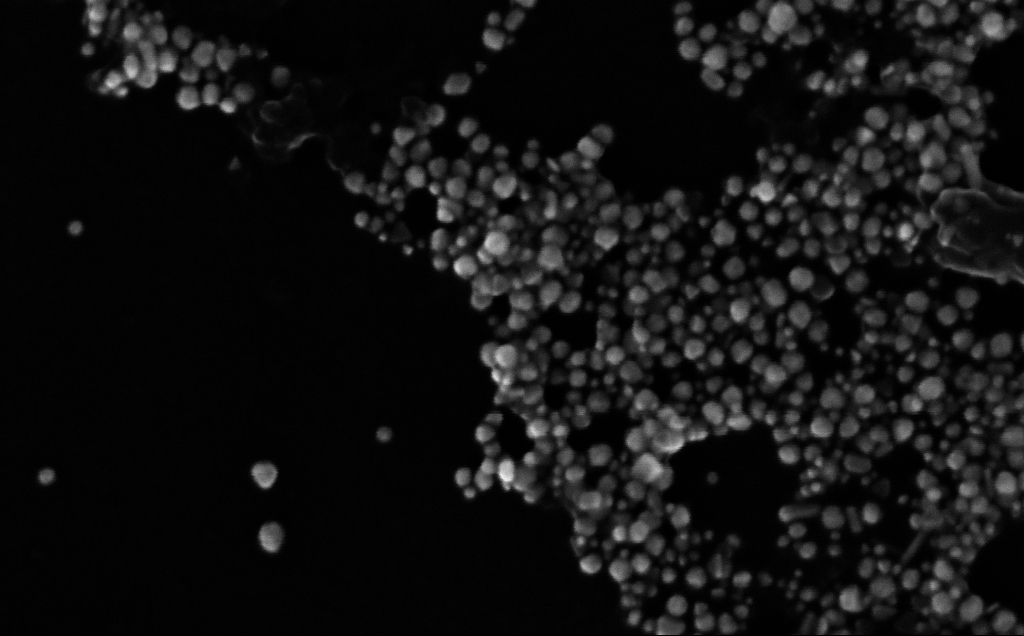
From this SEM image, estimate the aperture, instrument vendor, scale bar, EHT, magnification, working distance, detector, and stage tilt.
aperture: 30 µm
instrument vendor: Zeiss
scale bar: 200 nm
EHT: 10 kV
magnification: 344.81 K X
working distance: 4 mm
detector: InLens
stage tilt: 0°